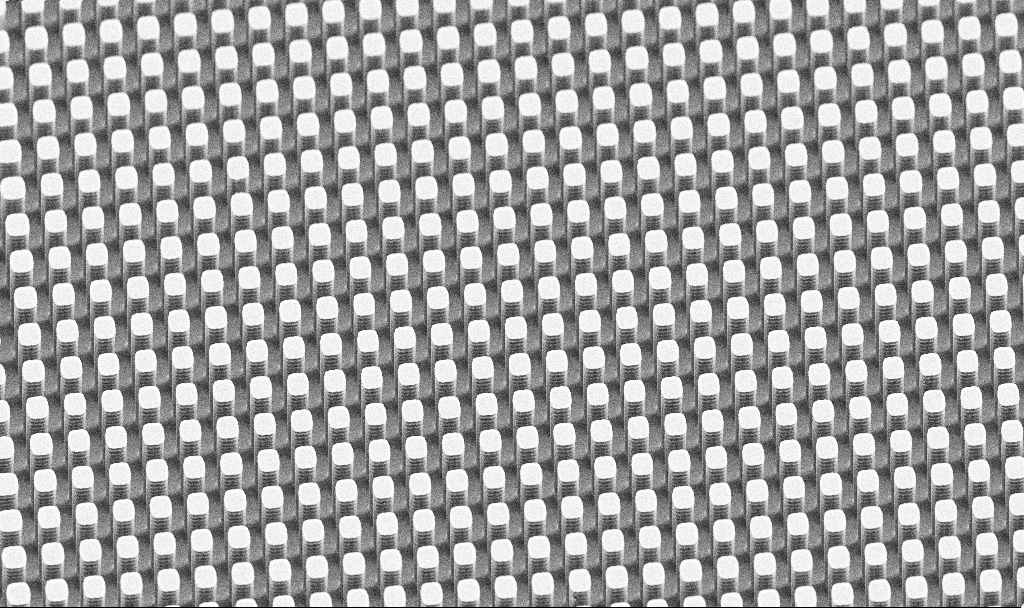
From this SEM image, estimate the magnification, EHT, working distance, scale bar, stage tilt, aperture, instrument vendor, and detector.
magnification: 3.42 K X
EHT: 5 kV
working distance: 7.6 mm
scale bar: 10000 nm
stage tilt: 45°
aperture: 30 µm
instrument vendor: Zeiss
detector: SE2